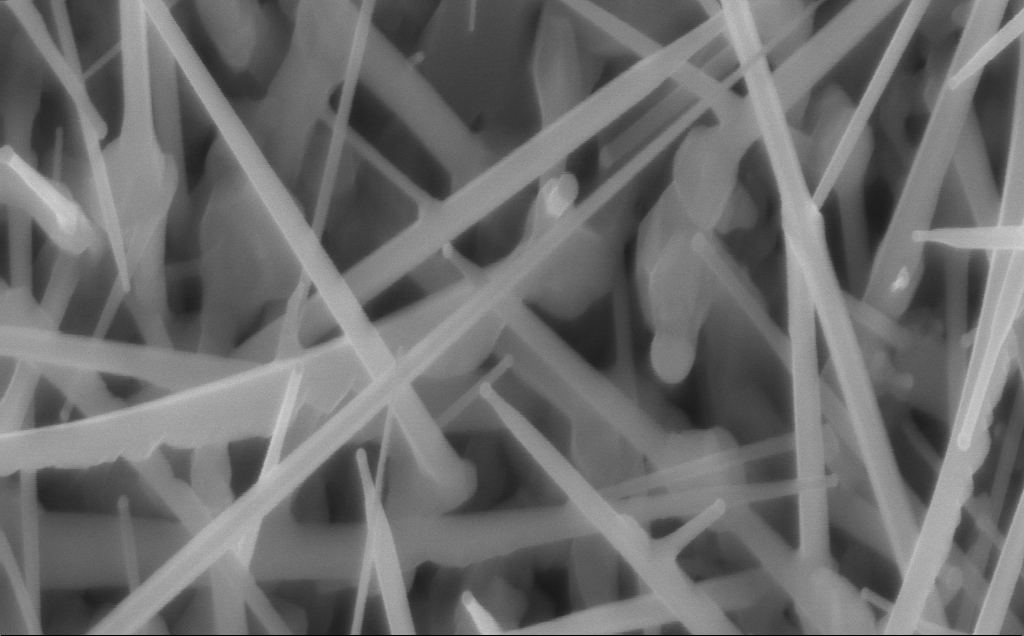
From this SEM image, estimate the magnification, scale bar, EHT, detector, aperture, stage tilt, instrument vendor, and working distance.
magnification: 80 K X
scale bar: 200 nm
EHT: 10 kV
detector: InLens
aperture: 30 µm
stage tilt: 0°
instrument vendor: Zeiss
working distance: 4 mm